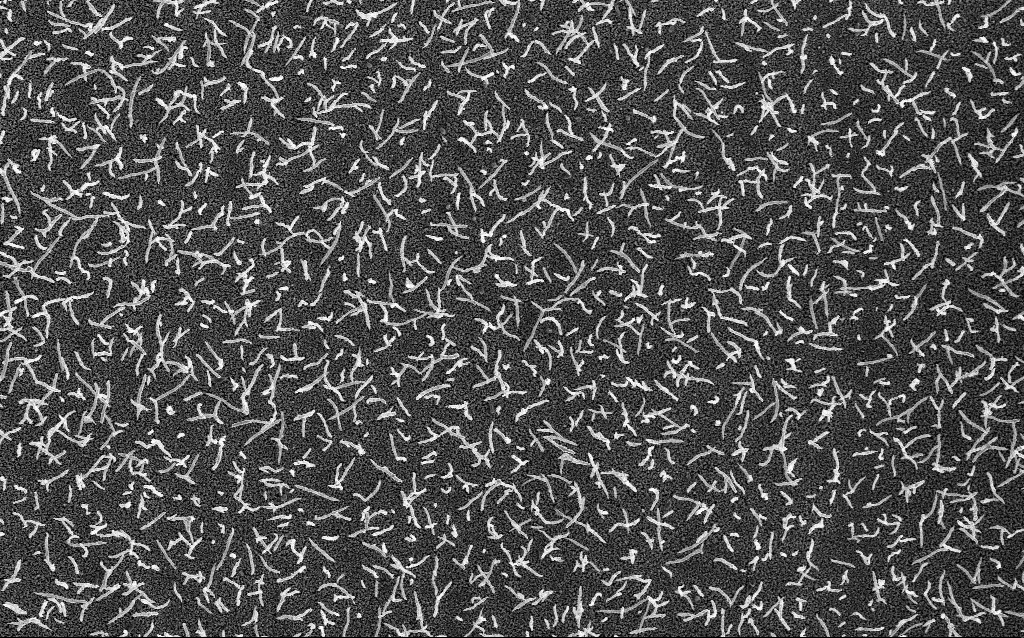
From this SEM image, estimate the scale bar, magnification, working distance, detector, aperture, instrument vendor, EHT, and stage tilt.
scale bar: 2000 nm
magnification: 10 K X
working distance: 1.8 mm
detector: InLens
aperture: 30 µm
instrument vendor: Zeiss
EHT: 5 kV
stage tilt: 0°